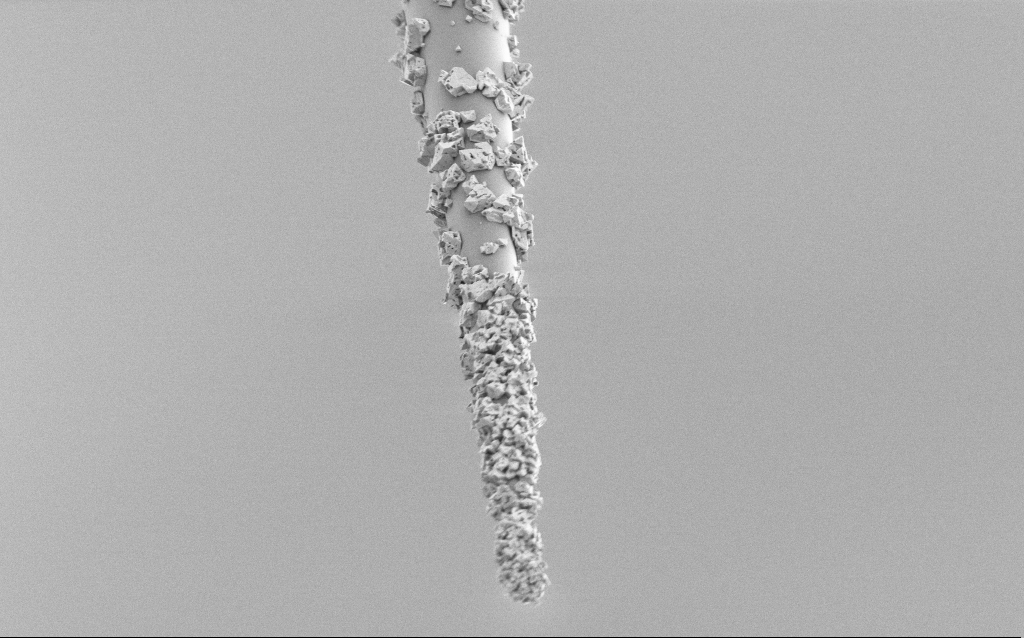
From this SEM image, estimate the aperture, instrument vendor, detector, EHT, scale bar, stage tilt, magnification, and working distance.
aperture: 30 µm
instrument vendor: Zeiss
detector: SE2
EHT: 1.5 kV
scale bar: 10000 nm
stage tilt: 45°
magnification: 5 K X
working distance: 7.7 mm